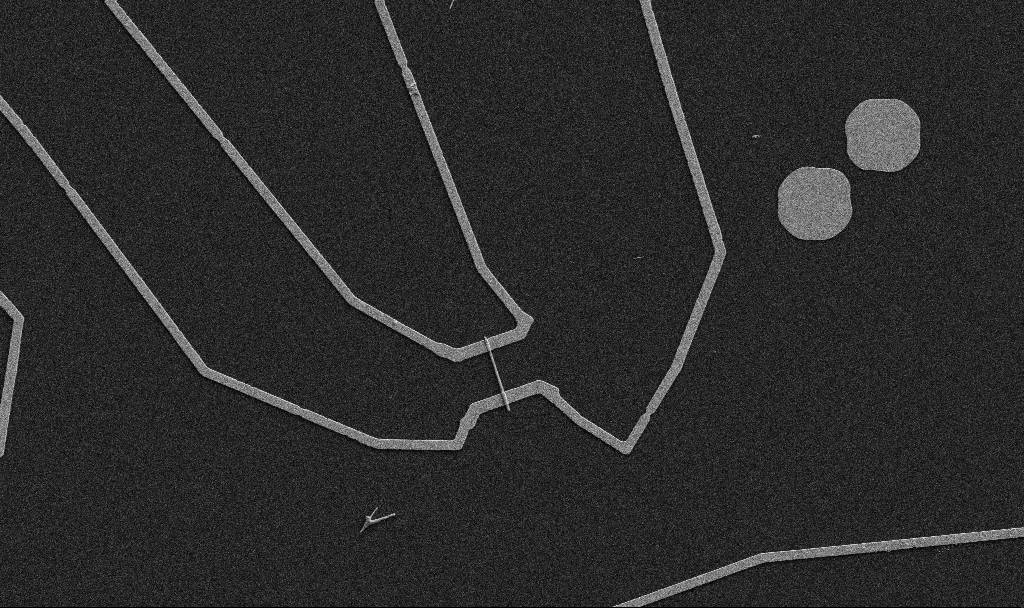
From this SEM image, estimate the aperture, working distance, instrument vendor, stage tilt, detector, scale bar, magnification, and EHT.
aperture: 30 µm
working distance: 10.7 mm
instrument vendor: Zeiss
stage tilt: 0°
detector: SE2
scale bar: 10000 nm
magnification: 5 K X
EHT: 5 kV